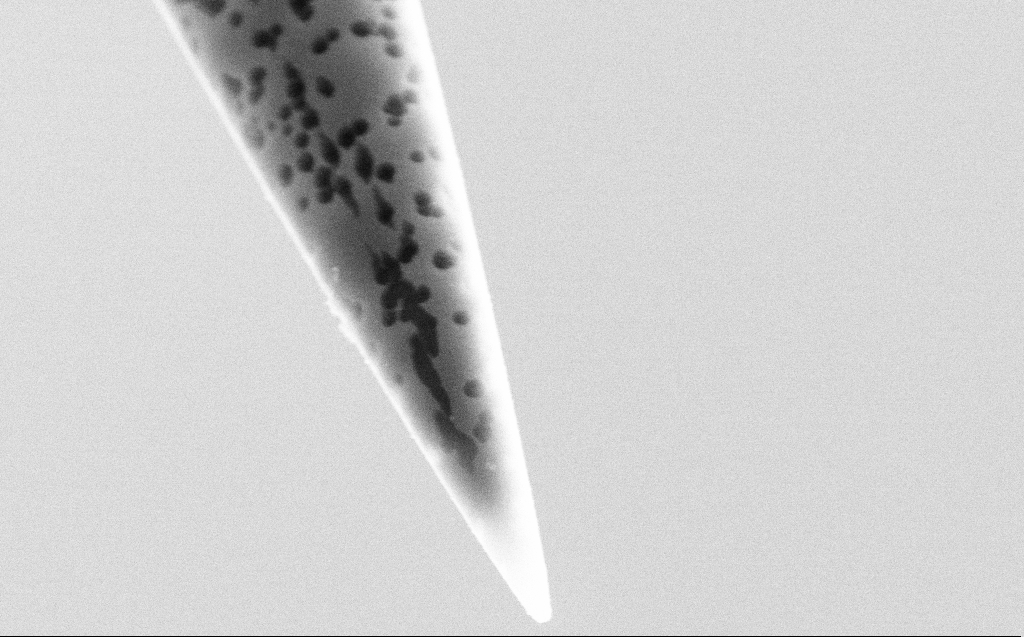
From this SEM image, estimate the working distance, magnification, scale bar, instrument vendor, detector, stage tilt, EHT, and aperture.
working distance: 6 mm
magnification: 50 K X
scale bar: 1000 nm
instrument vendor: Zeiss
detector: SE2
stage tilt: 45°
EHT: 5 kV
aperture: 30 µm